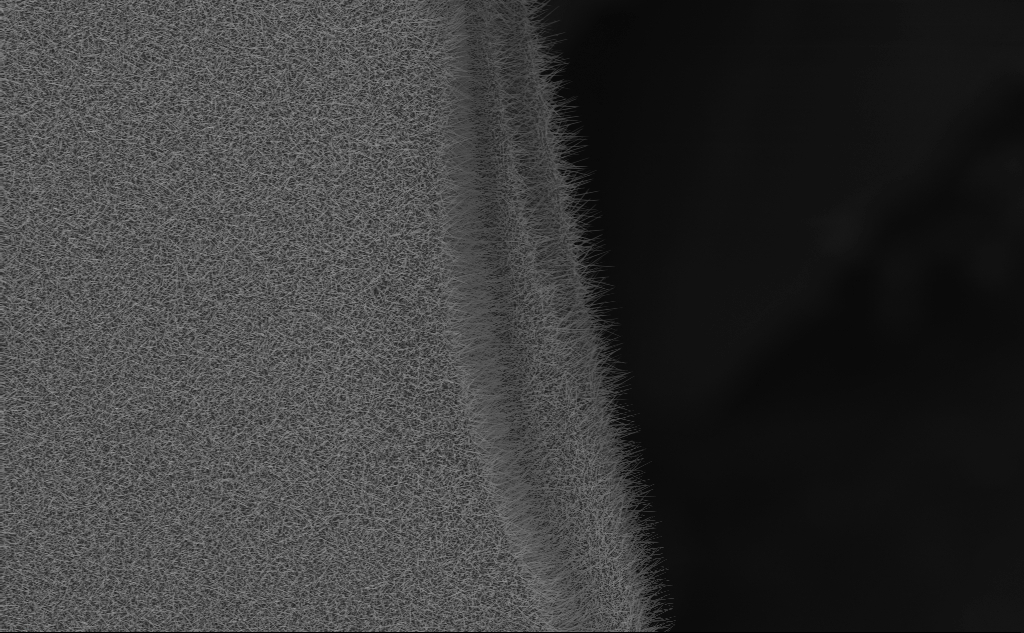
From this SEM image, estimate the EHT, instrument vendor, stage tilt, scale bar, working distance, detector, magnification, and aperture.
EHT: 10 kV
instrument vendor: Zeiss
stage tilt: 0°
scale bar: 10000 nm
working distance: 7 mm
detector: InLens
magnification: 2.48 K X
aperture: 30 µm